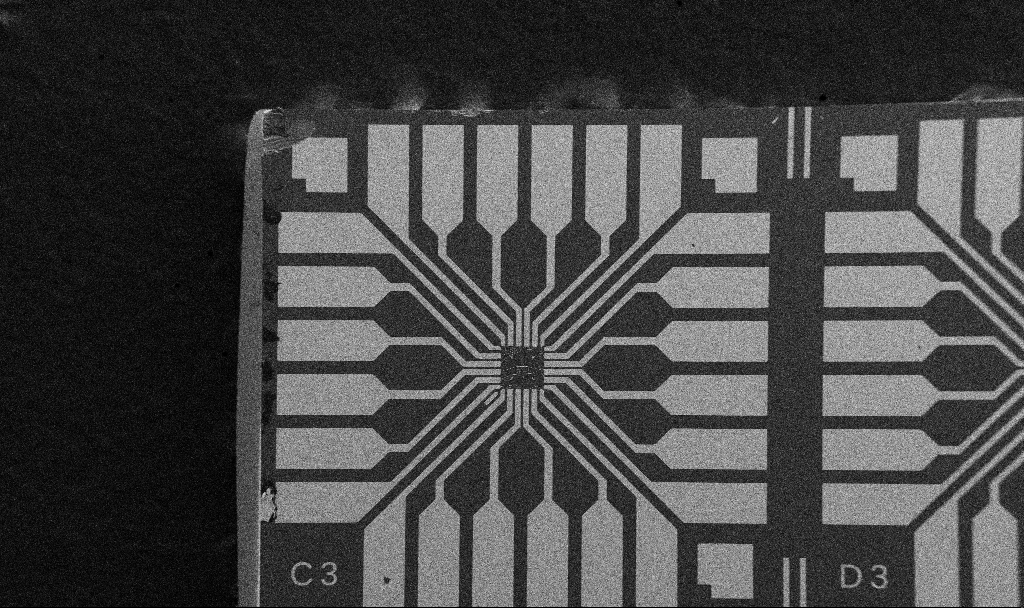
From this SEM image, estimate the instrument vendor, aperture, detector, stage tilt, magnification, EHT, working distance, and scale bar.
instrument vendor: Zeiss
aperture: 30 µm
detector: SE2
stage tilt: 0°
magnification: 0.1 K X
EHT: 5 kV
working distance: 10.7 mm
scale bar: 200000 nm